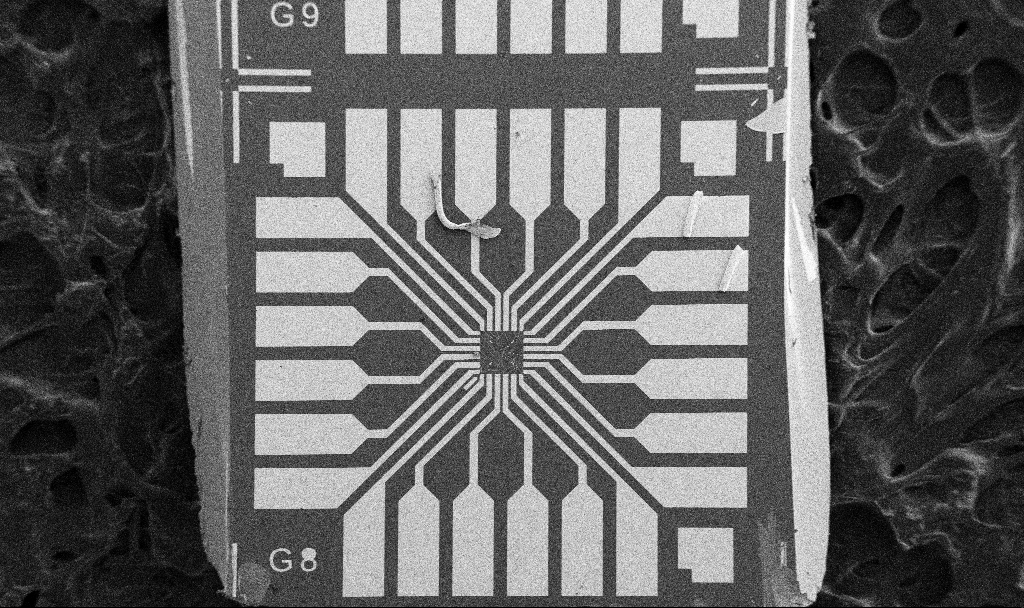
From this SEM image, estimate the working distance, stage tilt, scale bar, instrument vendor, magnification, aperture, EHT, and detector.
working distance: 10.7 mm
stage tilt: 0°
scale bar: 200000 nm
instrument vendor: Zeiss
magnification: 0.1 K X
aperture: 30 µm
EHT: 5 kV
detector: SE2